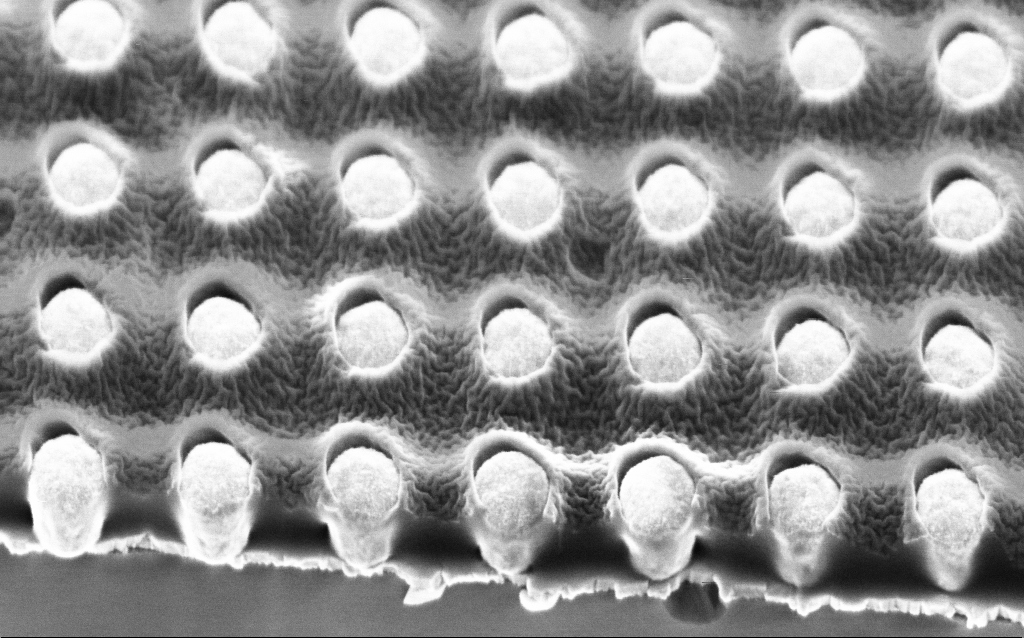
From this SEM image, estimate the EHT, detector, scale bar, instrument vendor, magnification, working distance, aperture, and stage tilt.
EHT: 2 kV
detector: InLens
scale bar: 200 nm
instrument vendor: Zeiss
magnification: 109.03 K X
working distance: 3.5 mm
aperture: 30 µm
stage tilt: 45°